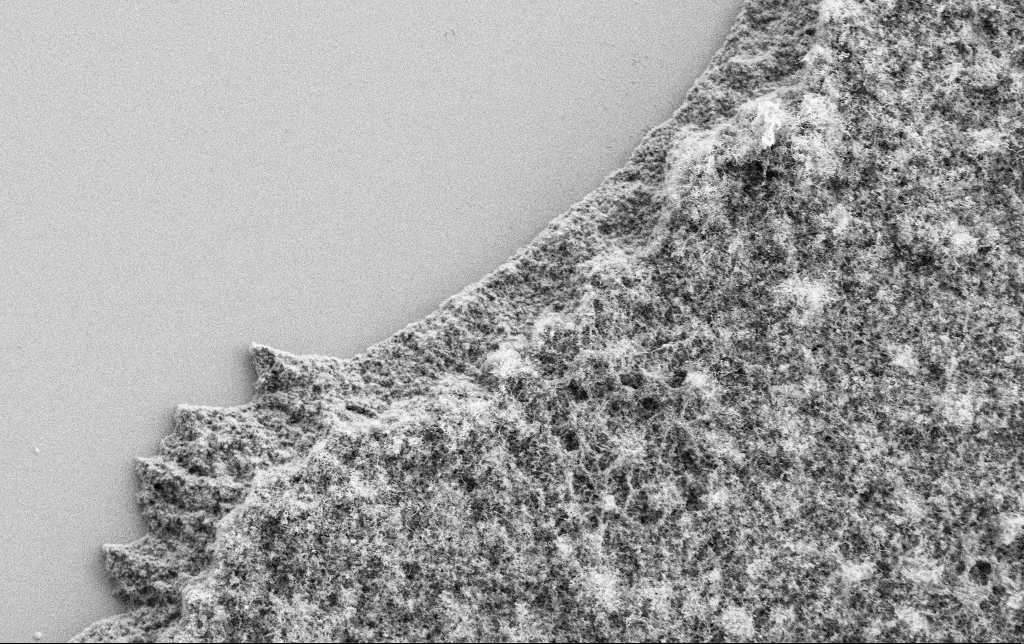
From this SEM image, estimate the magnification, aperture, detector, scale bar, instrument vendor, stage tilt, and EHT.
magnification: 2.5 K X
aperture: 30 µm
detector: SE2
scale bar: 10000 nm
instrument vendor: Zeiss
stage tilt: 0°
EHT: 2 kV